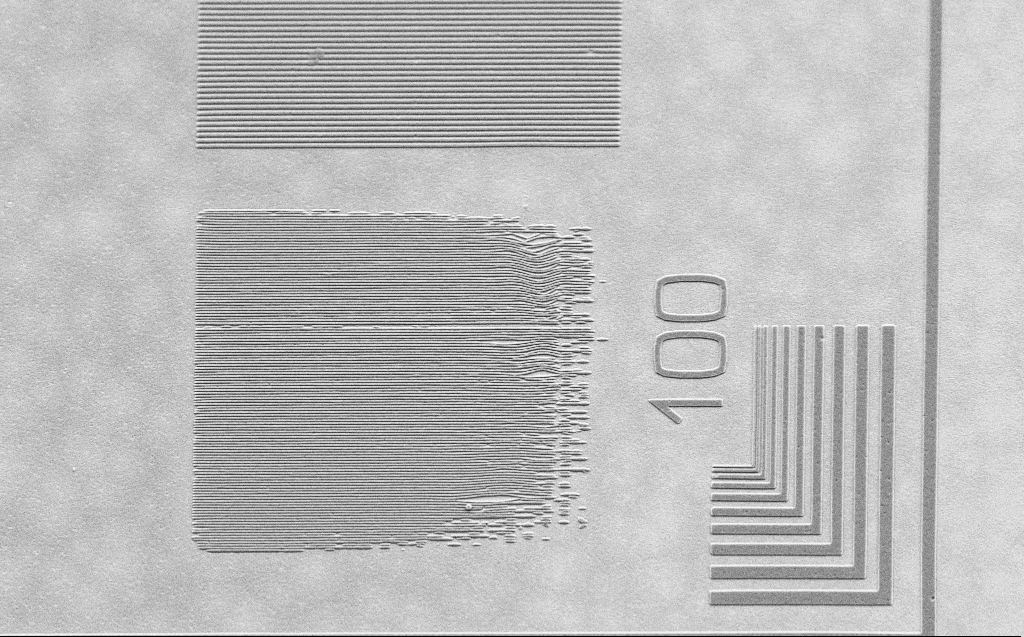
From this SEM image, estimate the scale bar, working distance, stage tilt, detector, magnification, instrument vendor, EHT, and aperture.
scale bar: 10000 nm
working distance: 5 mm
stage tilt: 30°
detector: SE2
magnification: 5.21 K X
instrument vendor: Zeiss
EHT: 2.5 kV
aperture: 30 µm